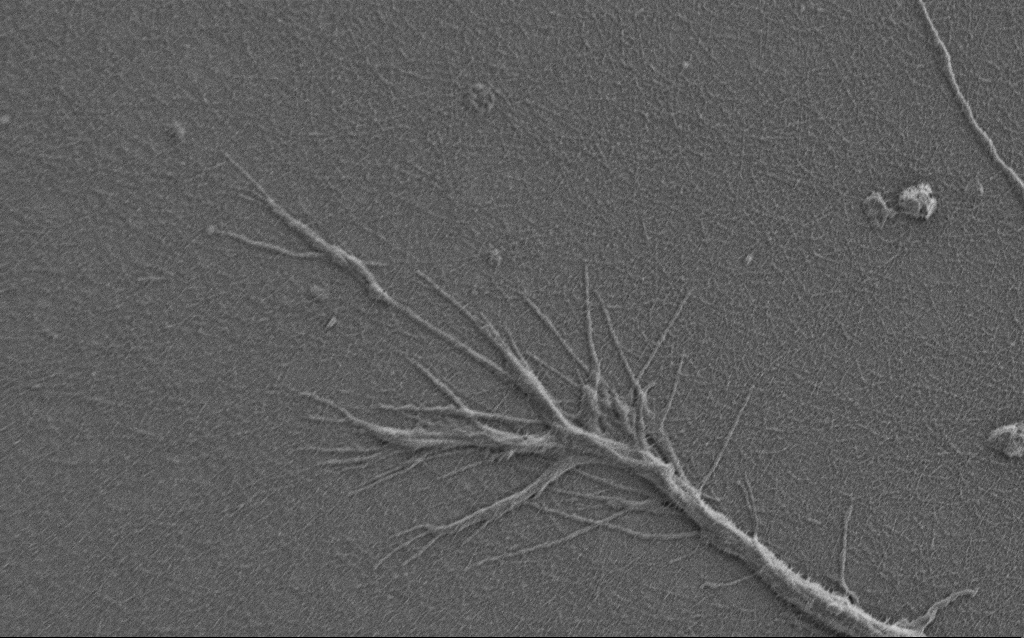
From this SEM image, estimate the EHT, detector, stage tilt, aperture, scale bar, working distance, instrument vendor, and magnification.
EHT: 0.9 kV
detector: SE2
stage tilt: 0°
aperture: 30 µm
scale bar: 2000 nm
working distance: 6 mm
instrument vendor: Zeiss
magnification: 10 K X